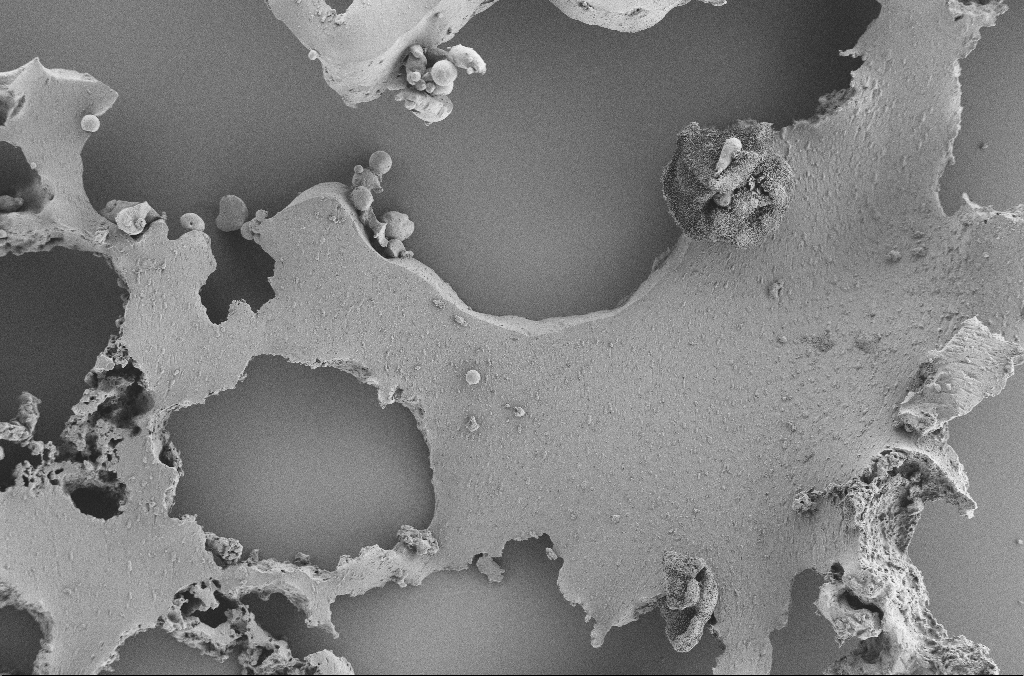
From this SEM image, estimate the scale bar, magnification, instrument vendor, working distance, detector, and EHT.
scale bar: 100000 nm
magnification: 0.25 K X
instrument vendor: Zeiss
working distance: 3.6 mm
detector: SE2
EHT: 2 kV